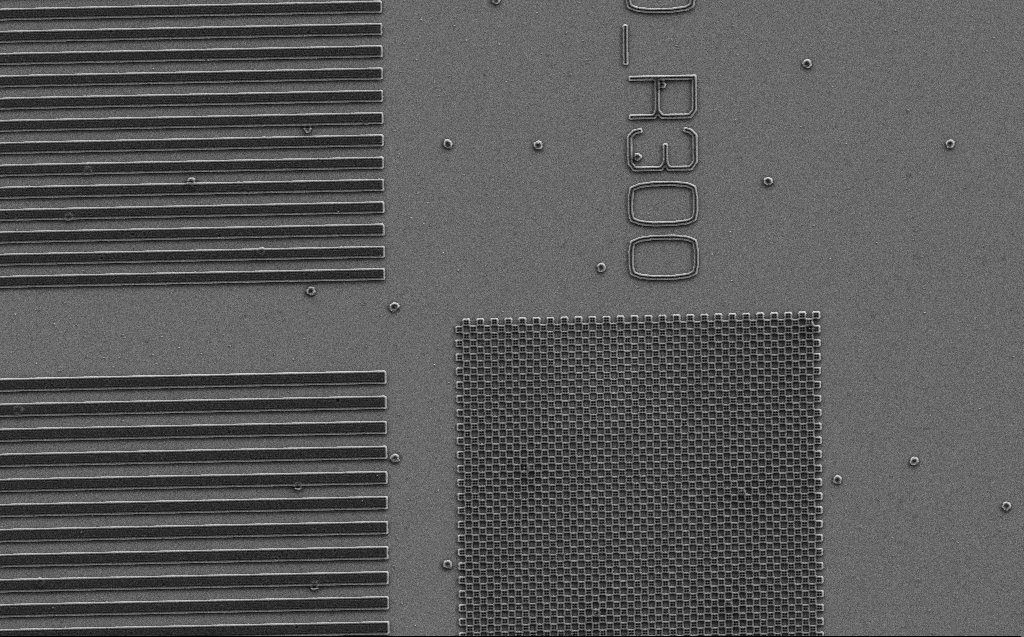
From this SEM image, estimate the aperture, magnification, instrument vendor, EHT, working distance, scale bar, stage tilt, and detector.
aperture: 30 µm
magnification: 5.19 K X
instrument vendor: Zeiss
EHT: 3 kV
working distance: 6 mm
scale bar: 10000 nm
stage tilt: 0°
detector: SE2